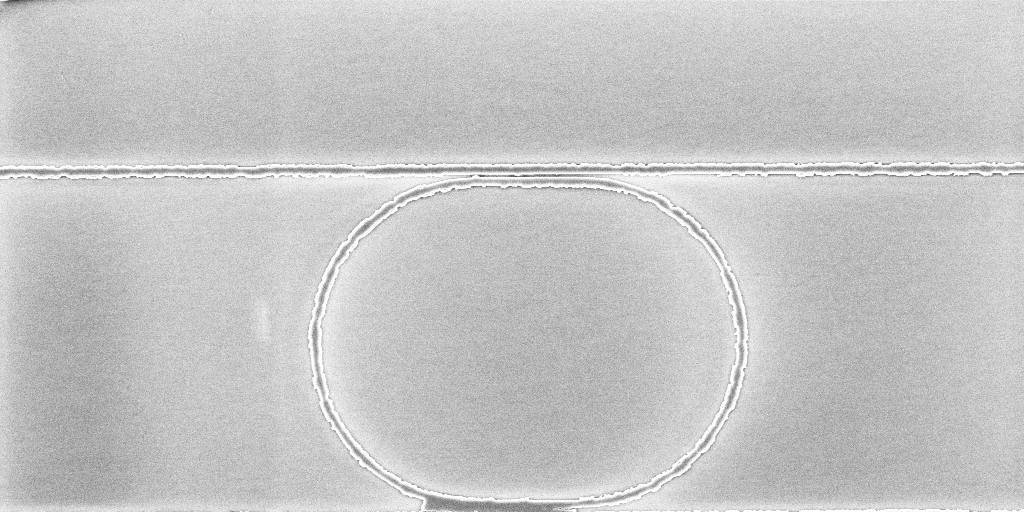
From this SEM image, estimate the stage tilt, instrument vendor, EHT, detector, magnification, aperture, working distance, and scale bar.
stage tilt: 0°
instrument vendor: Zeiss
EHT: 5 kV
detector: InLens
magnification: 6.19 K X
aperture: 30 µm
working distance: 10.1 mm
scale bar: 10000 nm